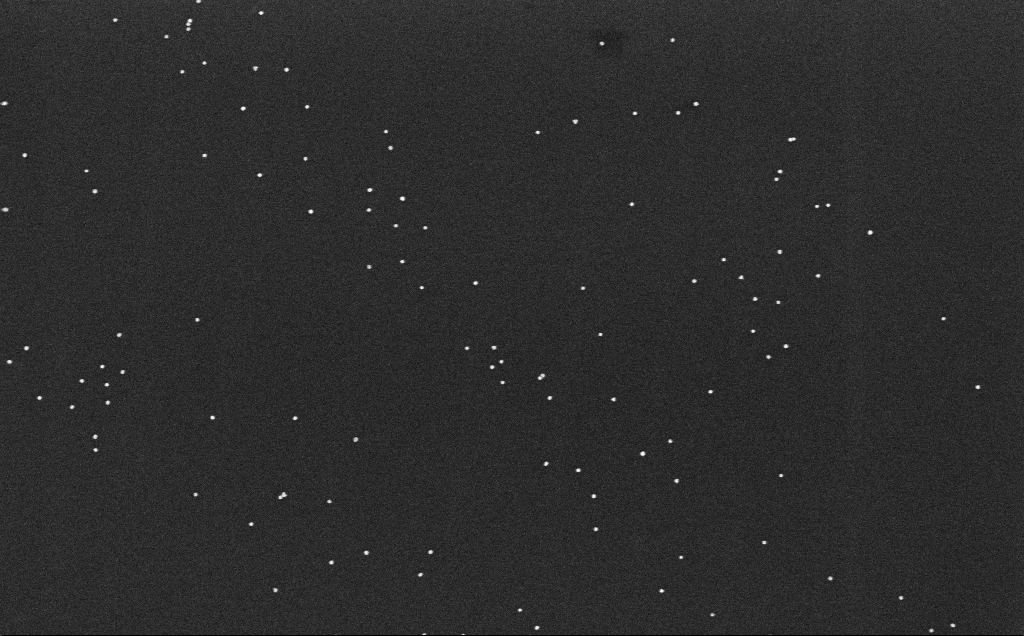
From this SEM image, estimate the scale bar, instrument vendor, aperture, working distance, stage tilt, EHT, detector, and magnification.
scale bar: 200 nm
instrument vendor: Zeiss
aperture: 30 µm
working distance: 6.6 mm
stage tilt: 0°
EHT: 10 kV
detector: InLens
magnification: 100 K X